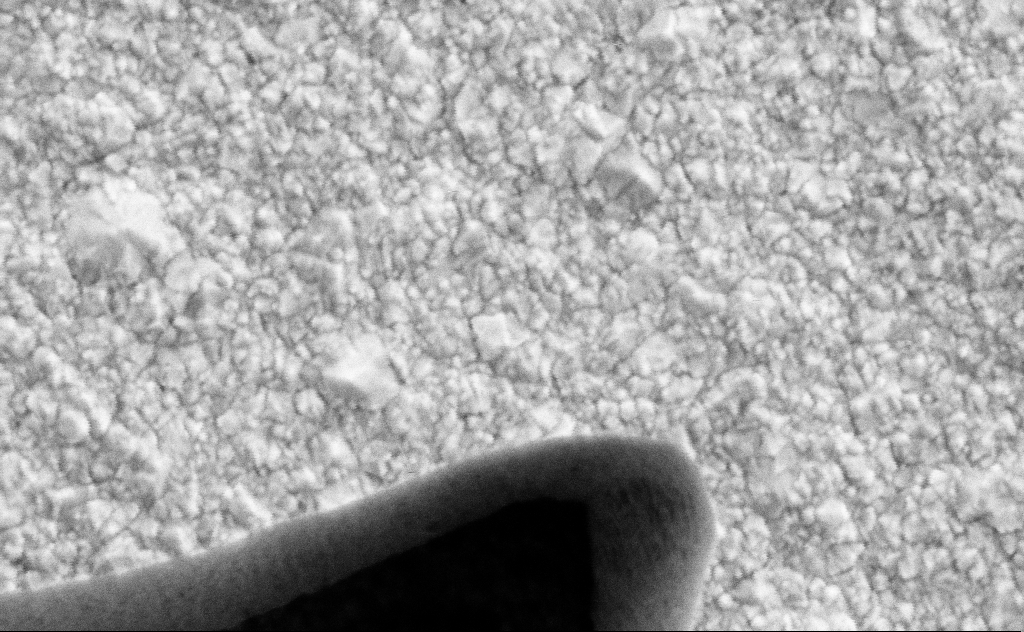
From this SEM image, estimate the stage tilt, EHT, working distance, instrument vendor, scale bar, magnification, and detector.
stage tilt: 0°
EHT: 3 kV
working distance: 14 mm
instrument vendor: Zeiss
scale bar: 200 nm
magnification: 95.72 K X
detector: SE2